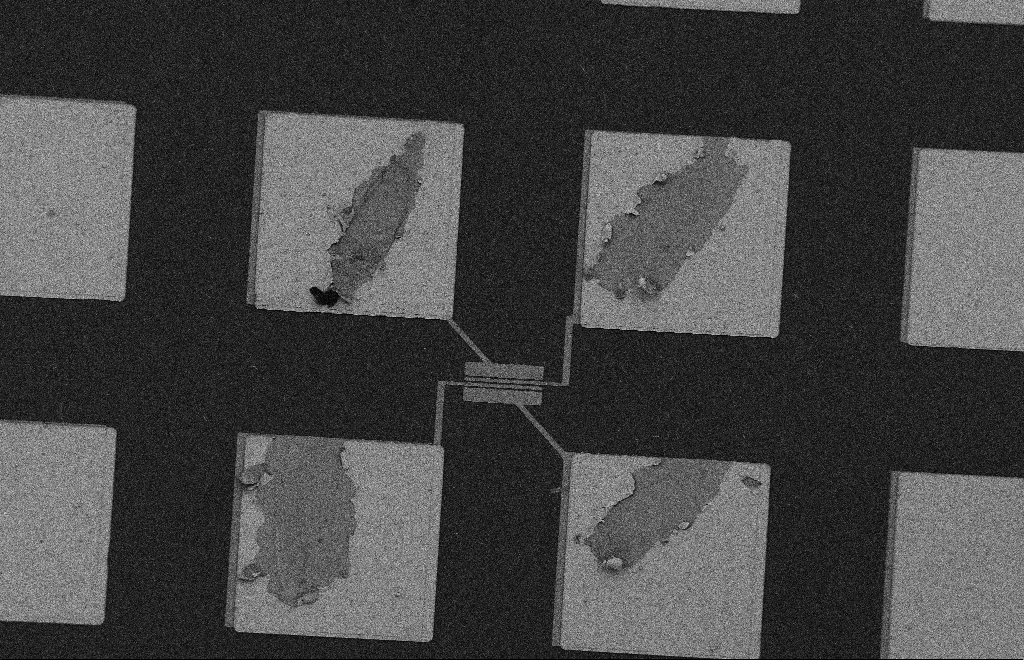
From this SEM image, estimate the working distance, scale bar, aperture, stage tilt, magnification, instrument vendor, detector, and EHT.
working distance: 10 mm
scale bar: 20000 nm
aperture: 20 µm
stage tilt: -0.3°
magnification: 0.474 K X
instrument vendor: Zeiss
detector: SE2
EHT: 2 kV